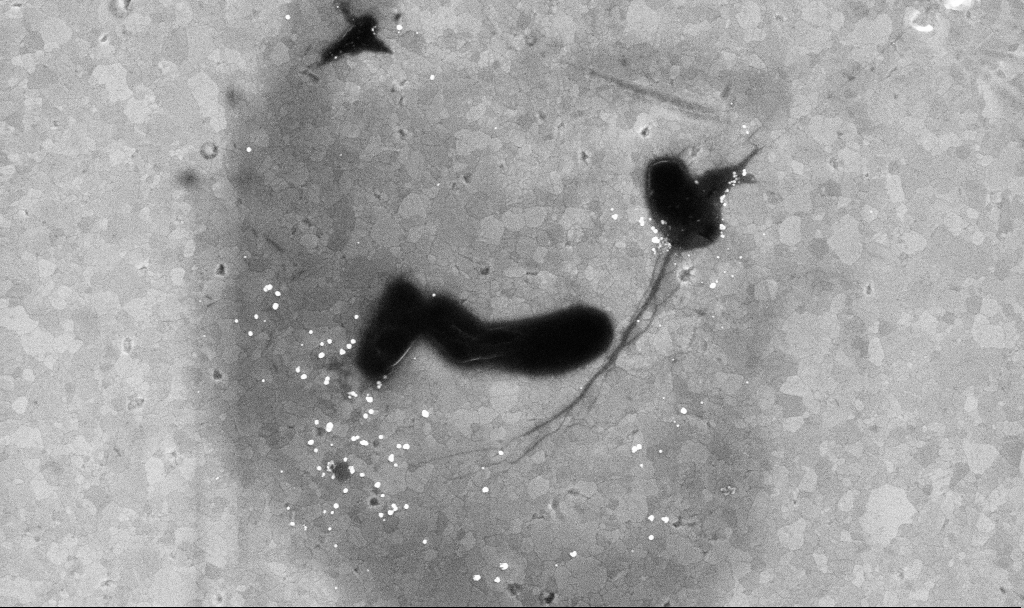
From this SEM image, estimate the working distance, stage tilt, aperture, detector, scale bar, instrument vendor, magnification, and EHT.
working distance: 3.4 mm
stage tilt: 0°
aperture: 30 µm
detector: InLens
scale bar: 1000 nm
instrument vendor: Zeiss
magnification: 30.95 K X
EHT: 10 kV